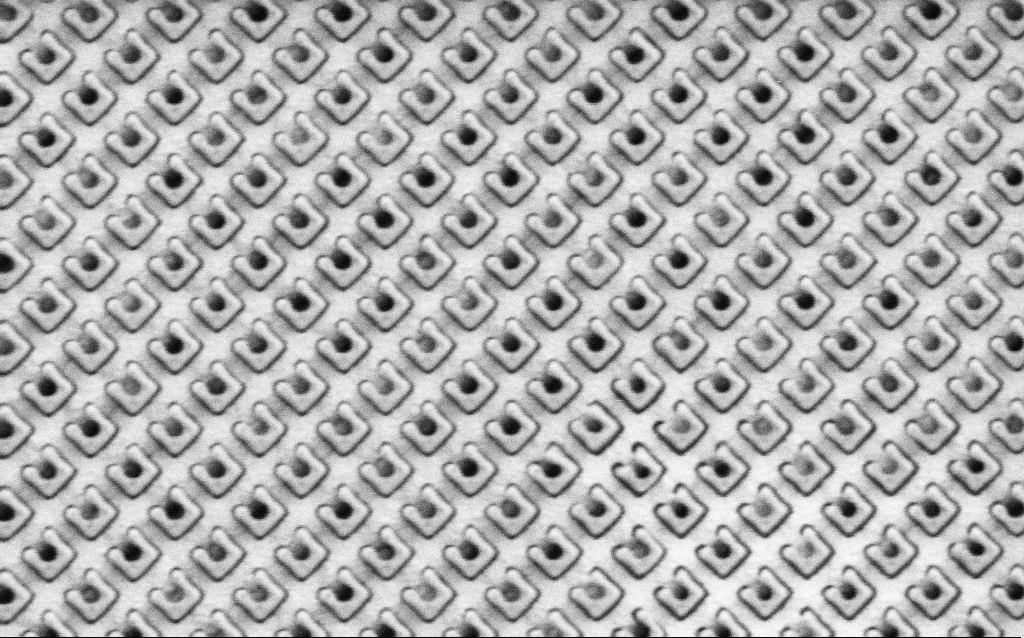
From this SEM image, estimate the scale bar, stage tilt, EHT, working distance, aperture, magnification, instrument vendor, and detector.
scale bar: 1000 nm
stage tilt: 0°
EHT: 1.5 kV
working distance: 8.1 mm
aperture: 30 µm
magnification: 46.81 K X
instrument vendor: Zeiss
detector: SE2